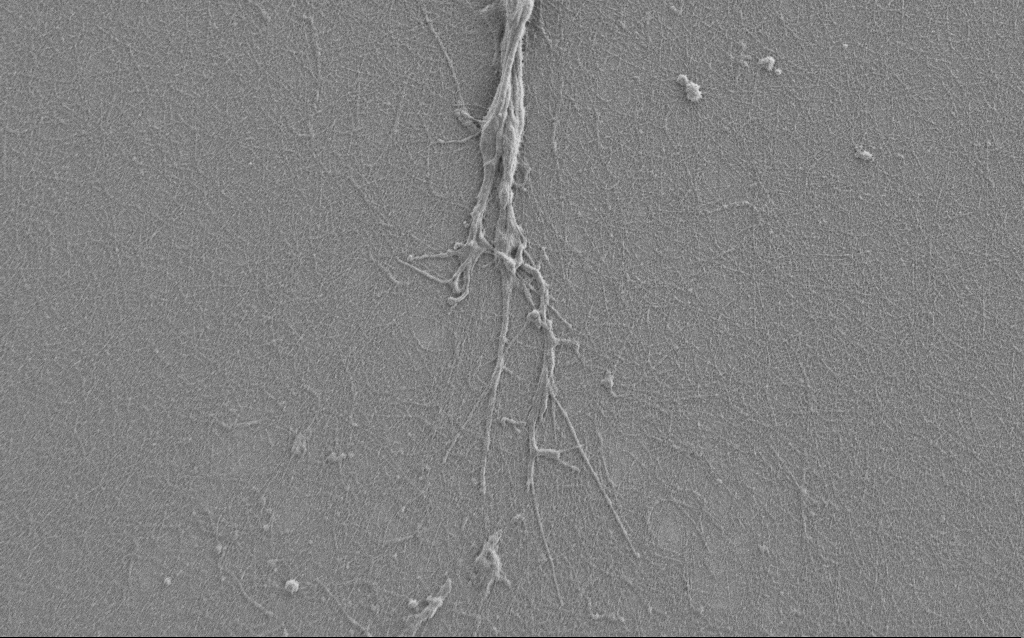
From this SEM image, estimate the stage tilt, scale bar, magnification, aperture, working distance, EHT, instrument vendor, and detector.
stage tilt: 0°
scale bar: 2000 nm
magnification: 7.5 K X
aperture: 30 µm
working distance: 6 mm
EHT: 1 kV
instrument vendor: Zeiss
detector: SE2